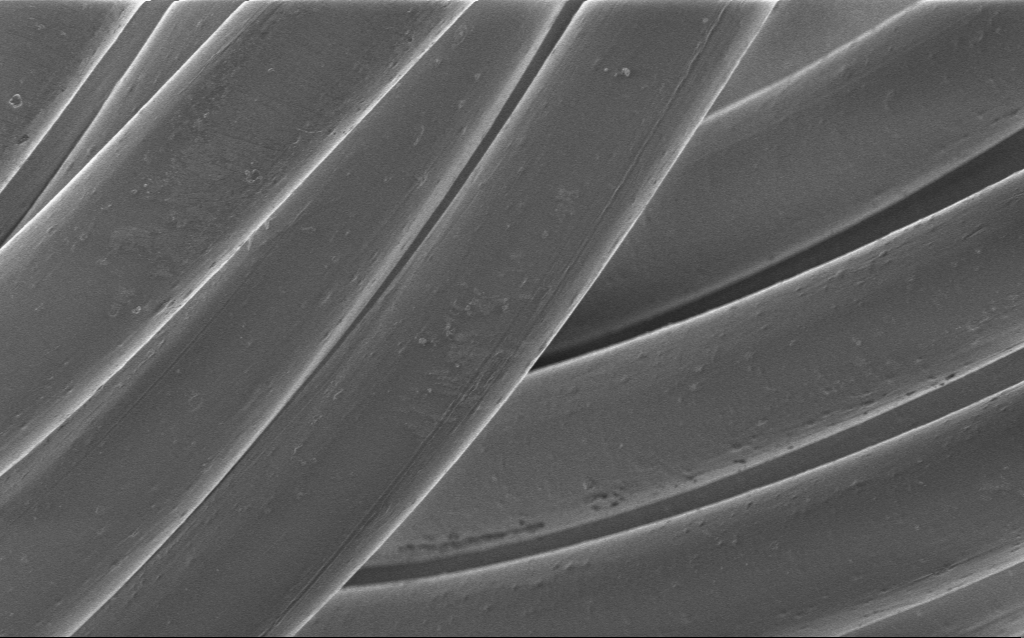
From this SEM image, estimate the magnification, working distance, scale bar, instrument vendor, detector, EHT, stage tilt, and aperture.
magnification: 2.57 K X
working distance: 4 mm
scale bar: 20000 nm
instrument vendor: Zeiss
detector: InLens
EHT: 1 kV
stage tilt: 0°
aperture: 30 µm